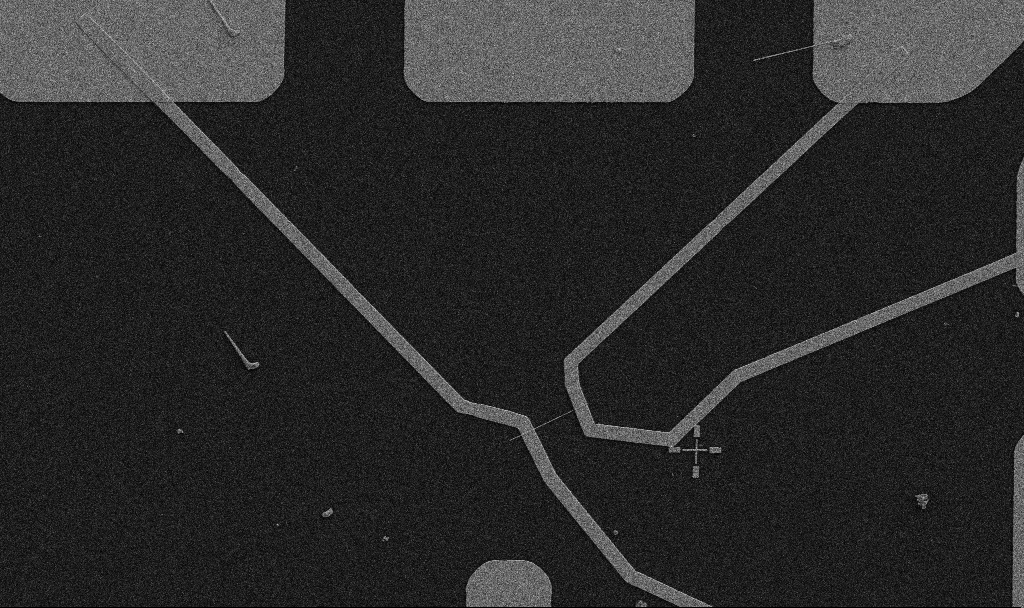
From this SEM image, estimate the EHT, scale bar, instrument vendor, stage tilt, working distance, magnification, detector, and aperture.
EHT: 5 kV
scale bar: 10000 nm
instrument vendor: Zeiss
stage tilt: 0°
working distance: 10.7 mm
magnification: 5 K X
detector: SE2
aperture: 30 µm